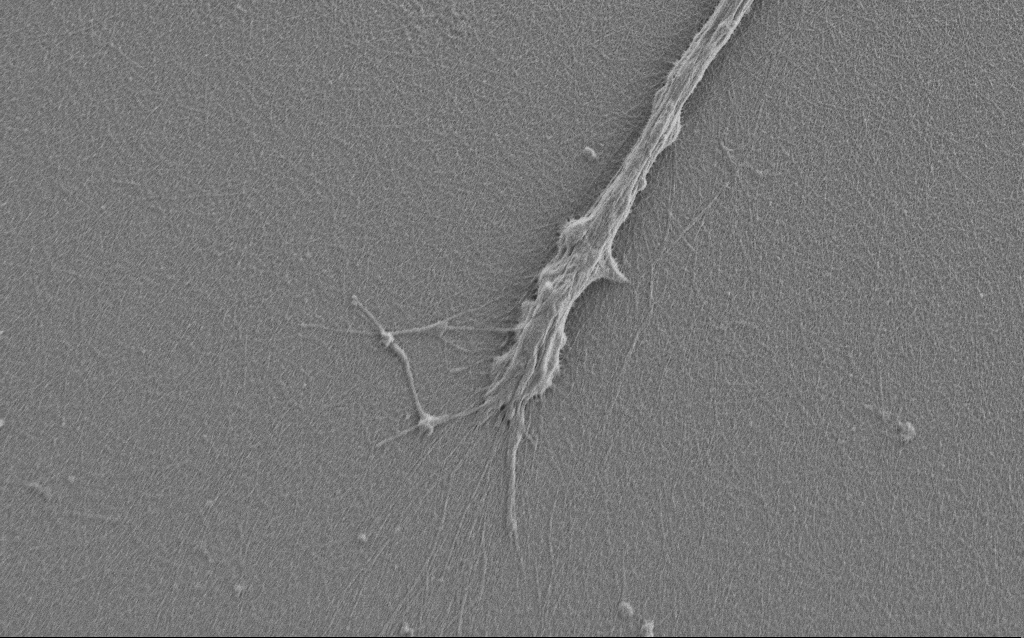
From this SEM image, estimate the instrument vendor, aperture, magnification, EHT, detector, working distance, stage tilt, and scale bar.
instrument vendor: Zeiss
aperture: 30 µm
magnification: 7.5 K X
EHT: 1 kV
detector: SE2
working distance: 6 mm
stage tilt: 0°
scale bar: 2000 nm